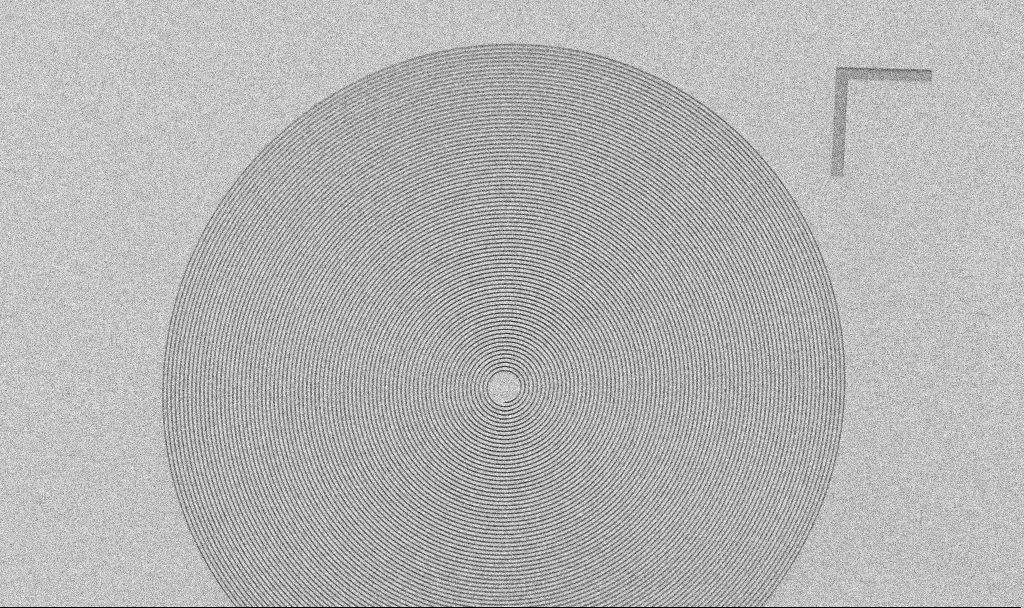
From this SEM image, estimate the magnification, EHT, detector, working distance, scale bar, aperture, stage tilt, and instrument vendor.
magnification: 2.36 K X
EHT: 5 kV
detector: SE2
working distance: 8.5 mm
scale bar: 10000 nm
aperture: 30 µm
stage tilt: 45°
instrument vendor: Zeiss